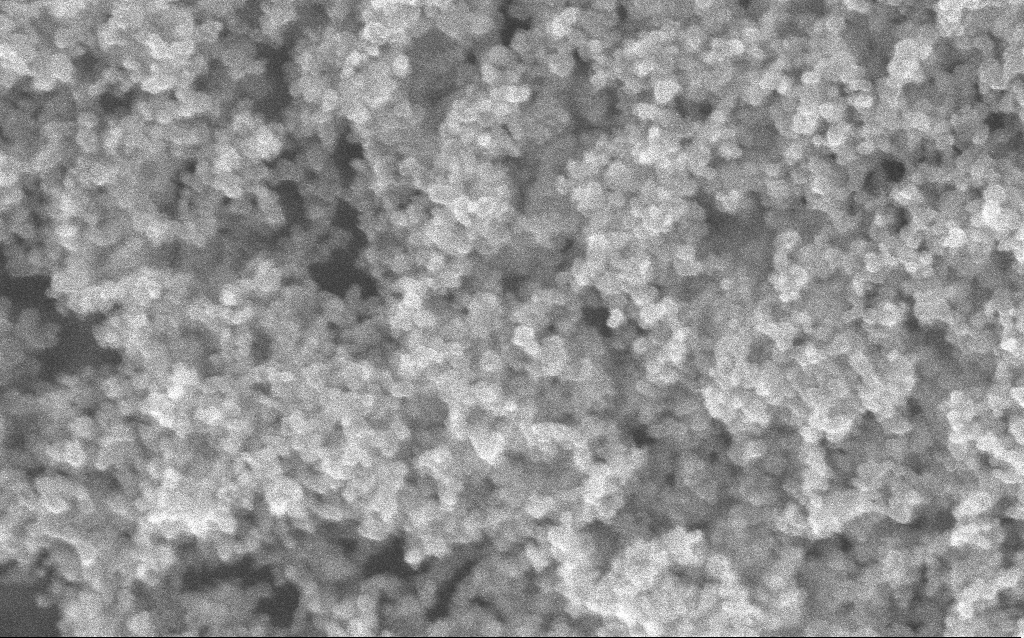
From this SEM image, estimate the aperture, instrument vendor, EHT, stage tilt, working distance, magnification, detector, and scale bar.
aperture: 30 µm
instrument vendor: Zeiss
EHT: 10 kV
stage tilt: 0°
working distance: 2.5 mm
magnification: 211.33 K X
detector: InLens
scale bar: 100 nm